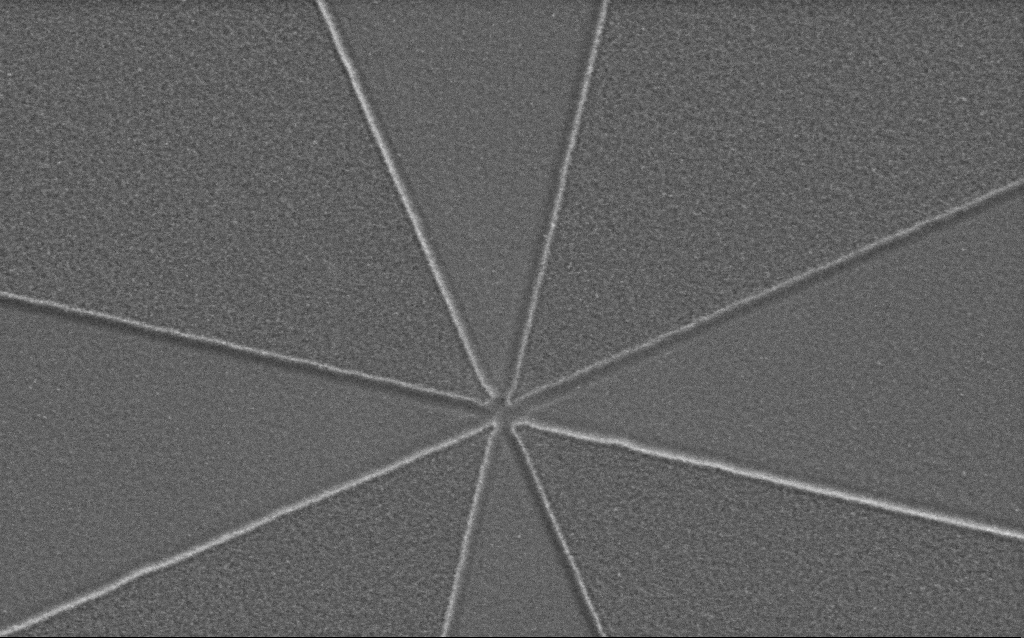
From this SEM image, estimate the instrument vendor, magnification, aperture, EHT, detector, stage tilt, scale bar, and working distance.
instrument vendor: Zeiss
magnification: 41.38 K X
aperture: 30 µm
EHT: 1.5 kV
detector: SE2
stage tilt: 0°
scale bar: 1000 nm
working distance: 5 mm